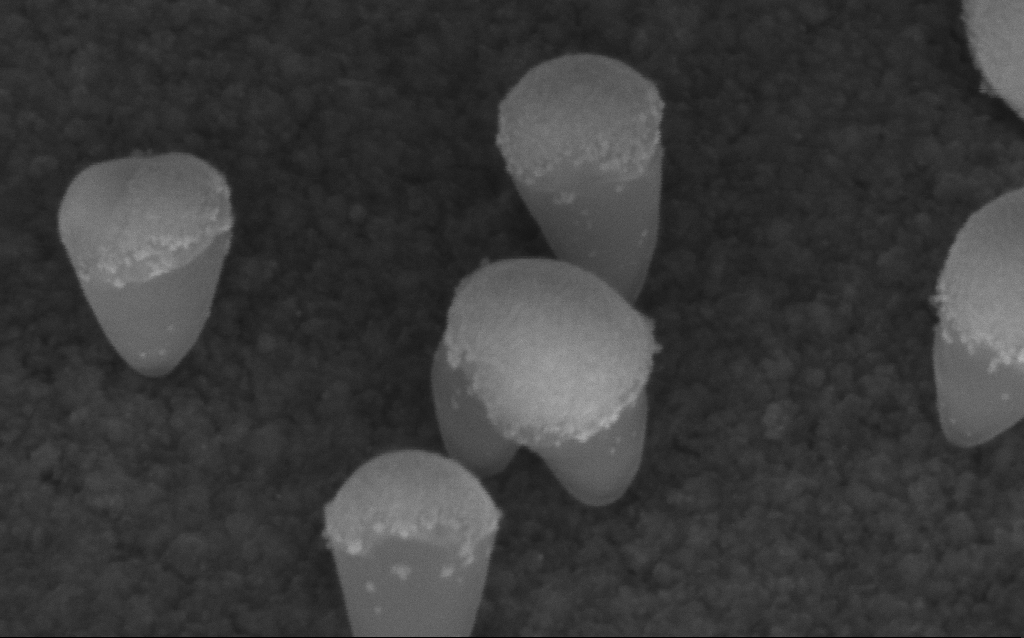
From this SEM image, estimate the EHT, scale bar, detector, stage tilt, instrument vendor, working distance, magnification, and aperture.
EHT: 5 kV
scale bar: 100 nm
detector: InLens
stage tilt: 45°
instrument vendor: Zeiss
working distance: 6.3 mm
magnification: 394.35 K X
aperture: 30 µm